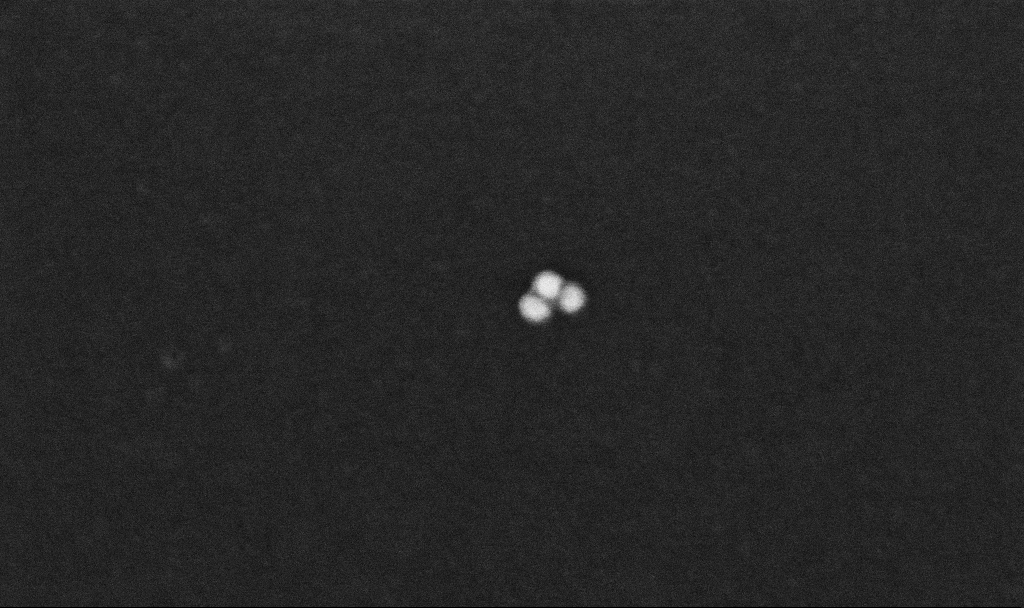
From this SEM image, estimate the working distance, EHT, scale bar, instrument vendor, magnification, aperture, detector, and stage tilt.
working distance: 3 mm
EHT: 10 kV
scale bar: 100 nm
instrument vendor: Zeiss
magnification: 424.33 K X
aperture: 30 µm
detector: InLens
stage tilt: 0°